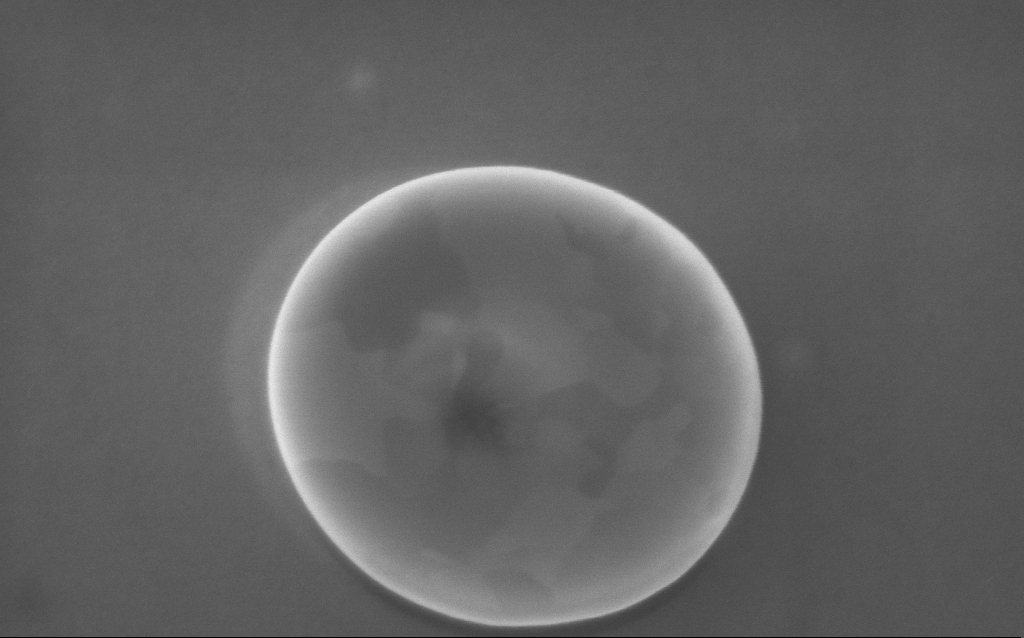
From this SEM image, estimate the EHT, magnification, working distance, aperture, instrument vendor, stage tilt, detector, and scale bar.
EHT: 5 kV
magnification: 105 K X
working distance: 3 mm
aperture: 30 µm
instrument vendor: Zeiss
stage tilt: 0°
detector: InLens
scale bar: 200 nm